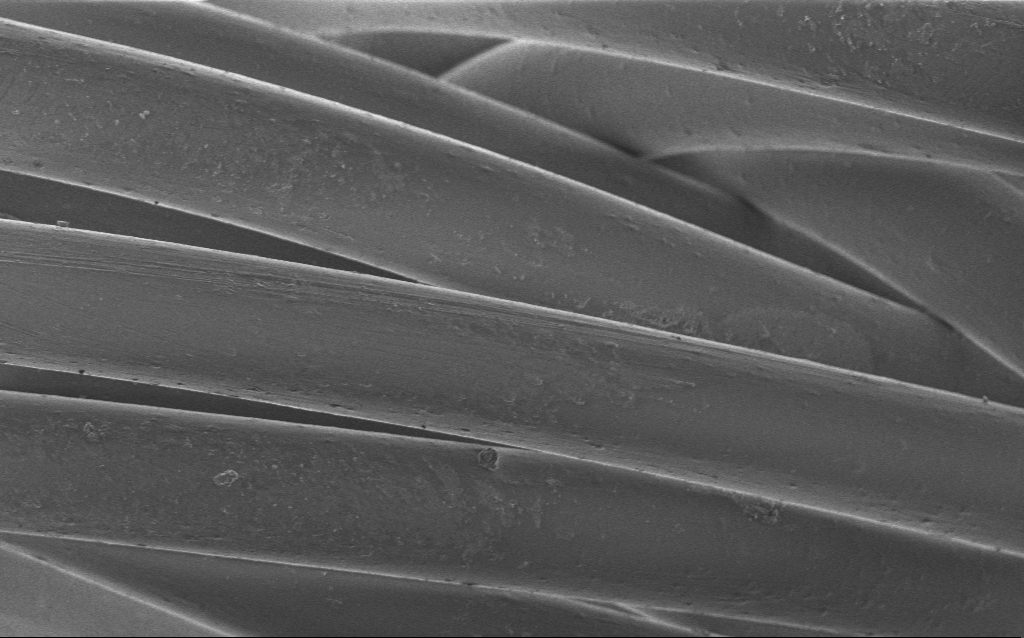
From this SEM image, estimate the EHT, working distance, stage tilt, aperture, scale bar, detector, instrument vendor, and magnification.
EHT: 1 kV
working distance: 4 mm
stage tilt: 0°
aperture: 30 µm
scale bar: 20000 nm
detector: InLens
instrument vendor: Zeiss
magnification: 2.28 K X